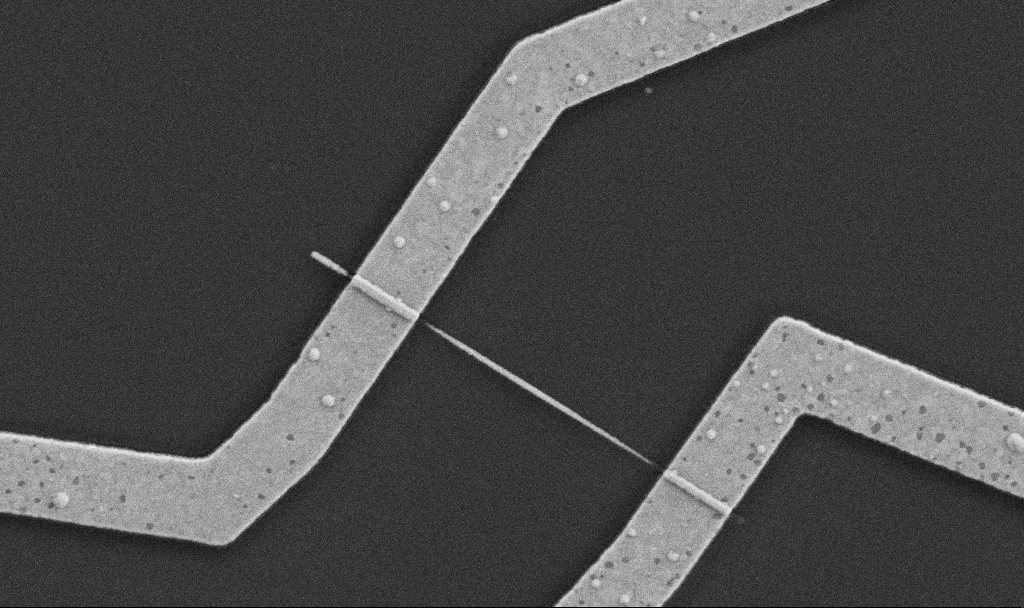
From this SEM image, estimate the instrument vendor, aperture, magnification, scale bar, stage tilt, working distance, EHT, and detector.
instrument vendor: Zeiss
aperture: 30 µm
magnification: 30 K X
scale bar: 1000 nm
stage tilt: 0°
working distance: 7.6 mm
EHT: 5 kV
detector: SE2